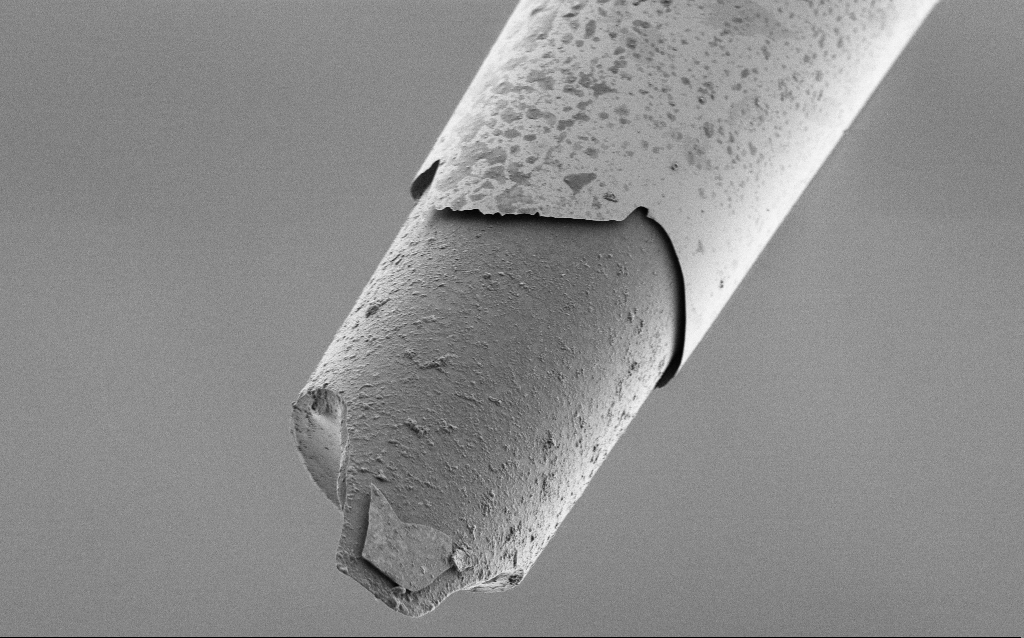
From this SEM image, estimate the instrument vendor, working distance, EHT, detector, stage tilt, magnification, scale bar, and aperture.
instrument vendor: Zeiss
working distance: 7.7 mm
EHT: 1 kV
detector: SE2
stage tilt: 45°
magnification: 2.5 K X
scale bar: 20000 nm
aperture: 30 µm